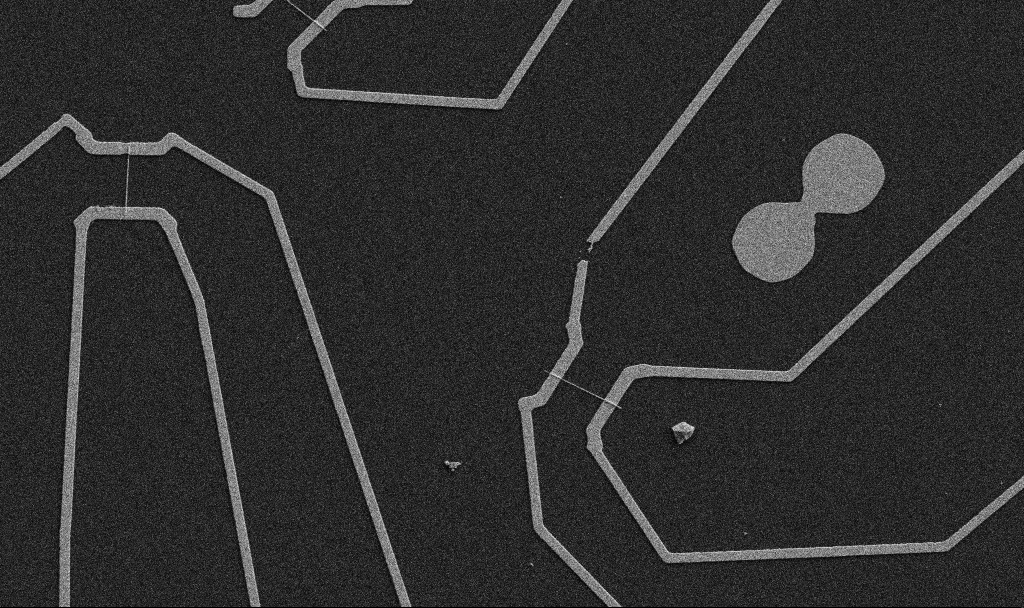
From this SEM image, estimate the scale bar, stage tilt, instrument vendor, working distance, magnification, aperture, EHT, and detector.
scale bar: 10000 nm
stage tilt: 0°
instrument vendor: Zeiss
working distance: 10.7 mm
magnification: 5 K X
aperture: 30 µm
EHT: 5 kV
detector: SE2